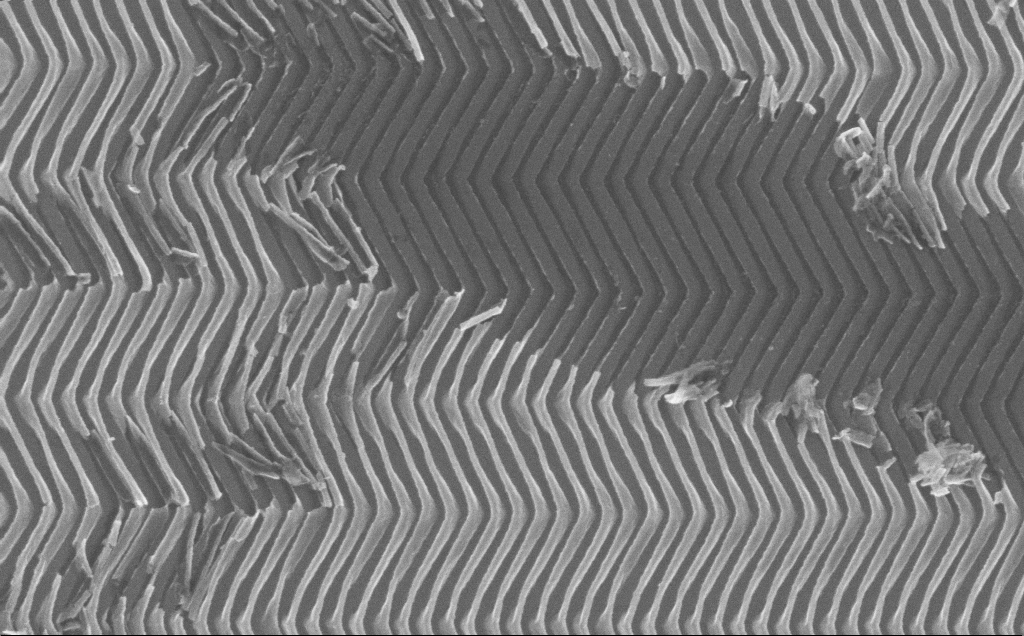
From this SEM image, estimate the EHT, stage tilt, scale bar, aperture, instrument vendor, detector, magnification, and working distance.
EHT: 10 kV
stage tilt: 0°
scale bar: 1000 nm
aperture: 30 µm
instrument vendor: Zeiss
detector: InLens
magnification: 41.24 K X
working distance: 7 mm